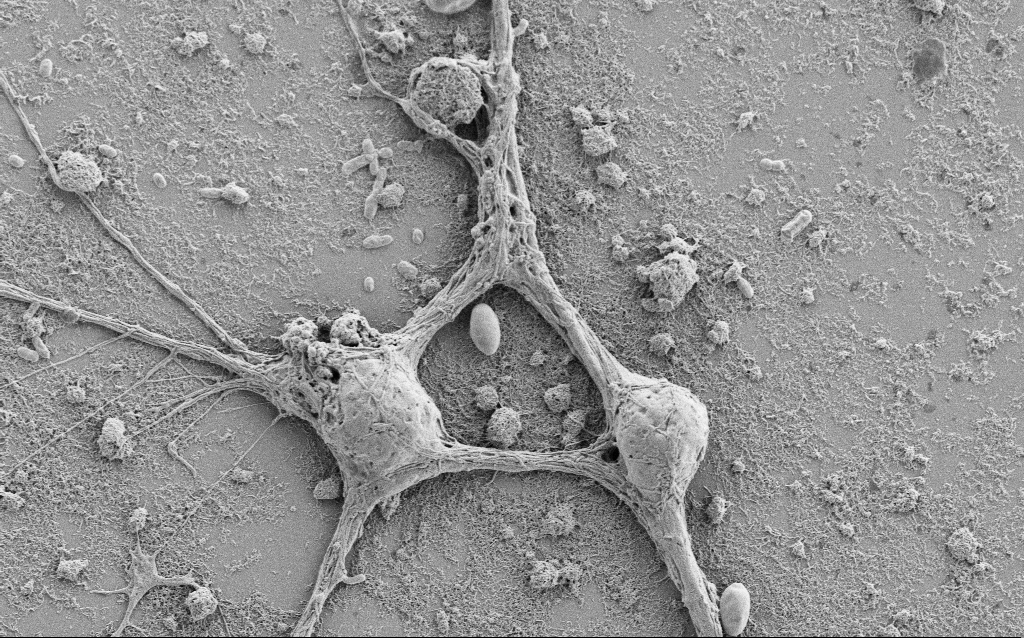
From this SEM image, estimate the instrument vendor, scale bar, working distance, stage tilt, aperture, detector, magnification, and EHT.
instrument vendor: Zeiss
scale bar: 10000 nm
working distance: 6.9 mm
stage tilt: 0°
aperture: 30 µm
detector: SE2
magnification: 5 K X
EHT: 1.5 kV